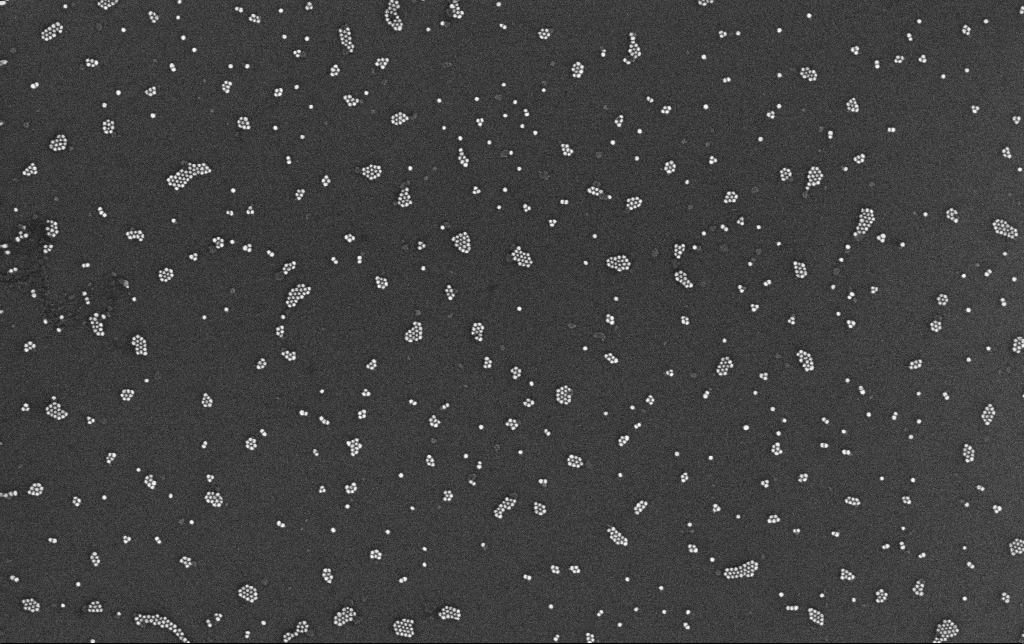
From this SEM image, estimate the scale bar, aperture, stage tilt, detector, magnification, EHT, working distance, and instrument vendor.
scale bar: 200 nm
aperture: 30 µm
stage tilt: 0°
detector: InLens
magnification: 100 K X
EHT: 10 kV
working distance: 3.4 mm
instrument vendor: Zeiss